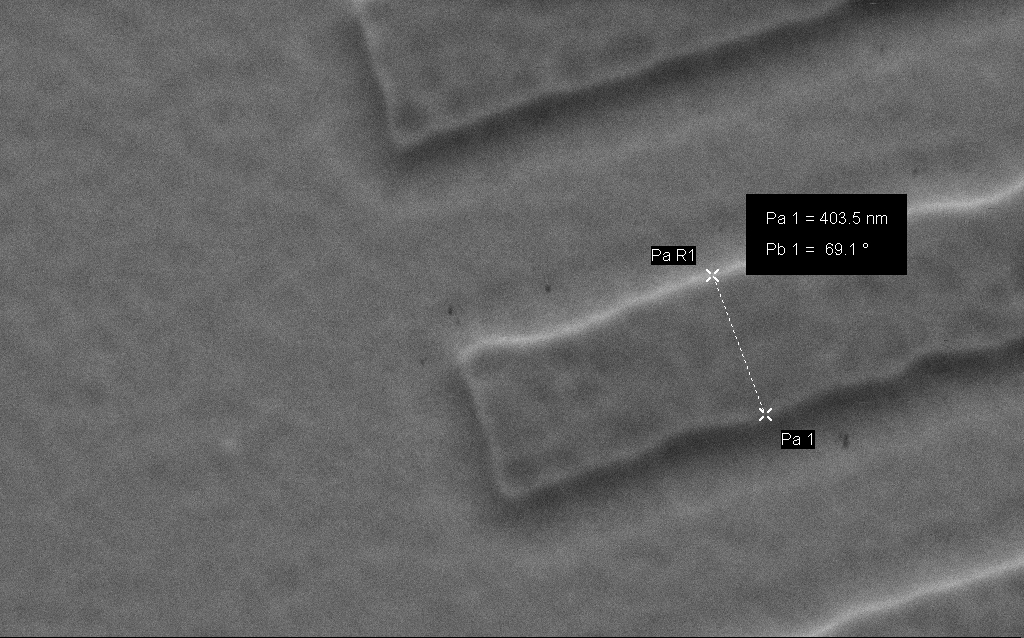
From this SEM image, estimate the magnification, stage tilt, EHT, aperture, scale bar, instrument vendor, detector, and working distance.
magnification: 135.39 K X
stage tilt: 45°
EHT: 2 kV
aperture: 30 µm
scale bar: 200 nm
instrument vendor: Zeiss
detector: SE2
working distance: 4 mm